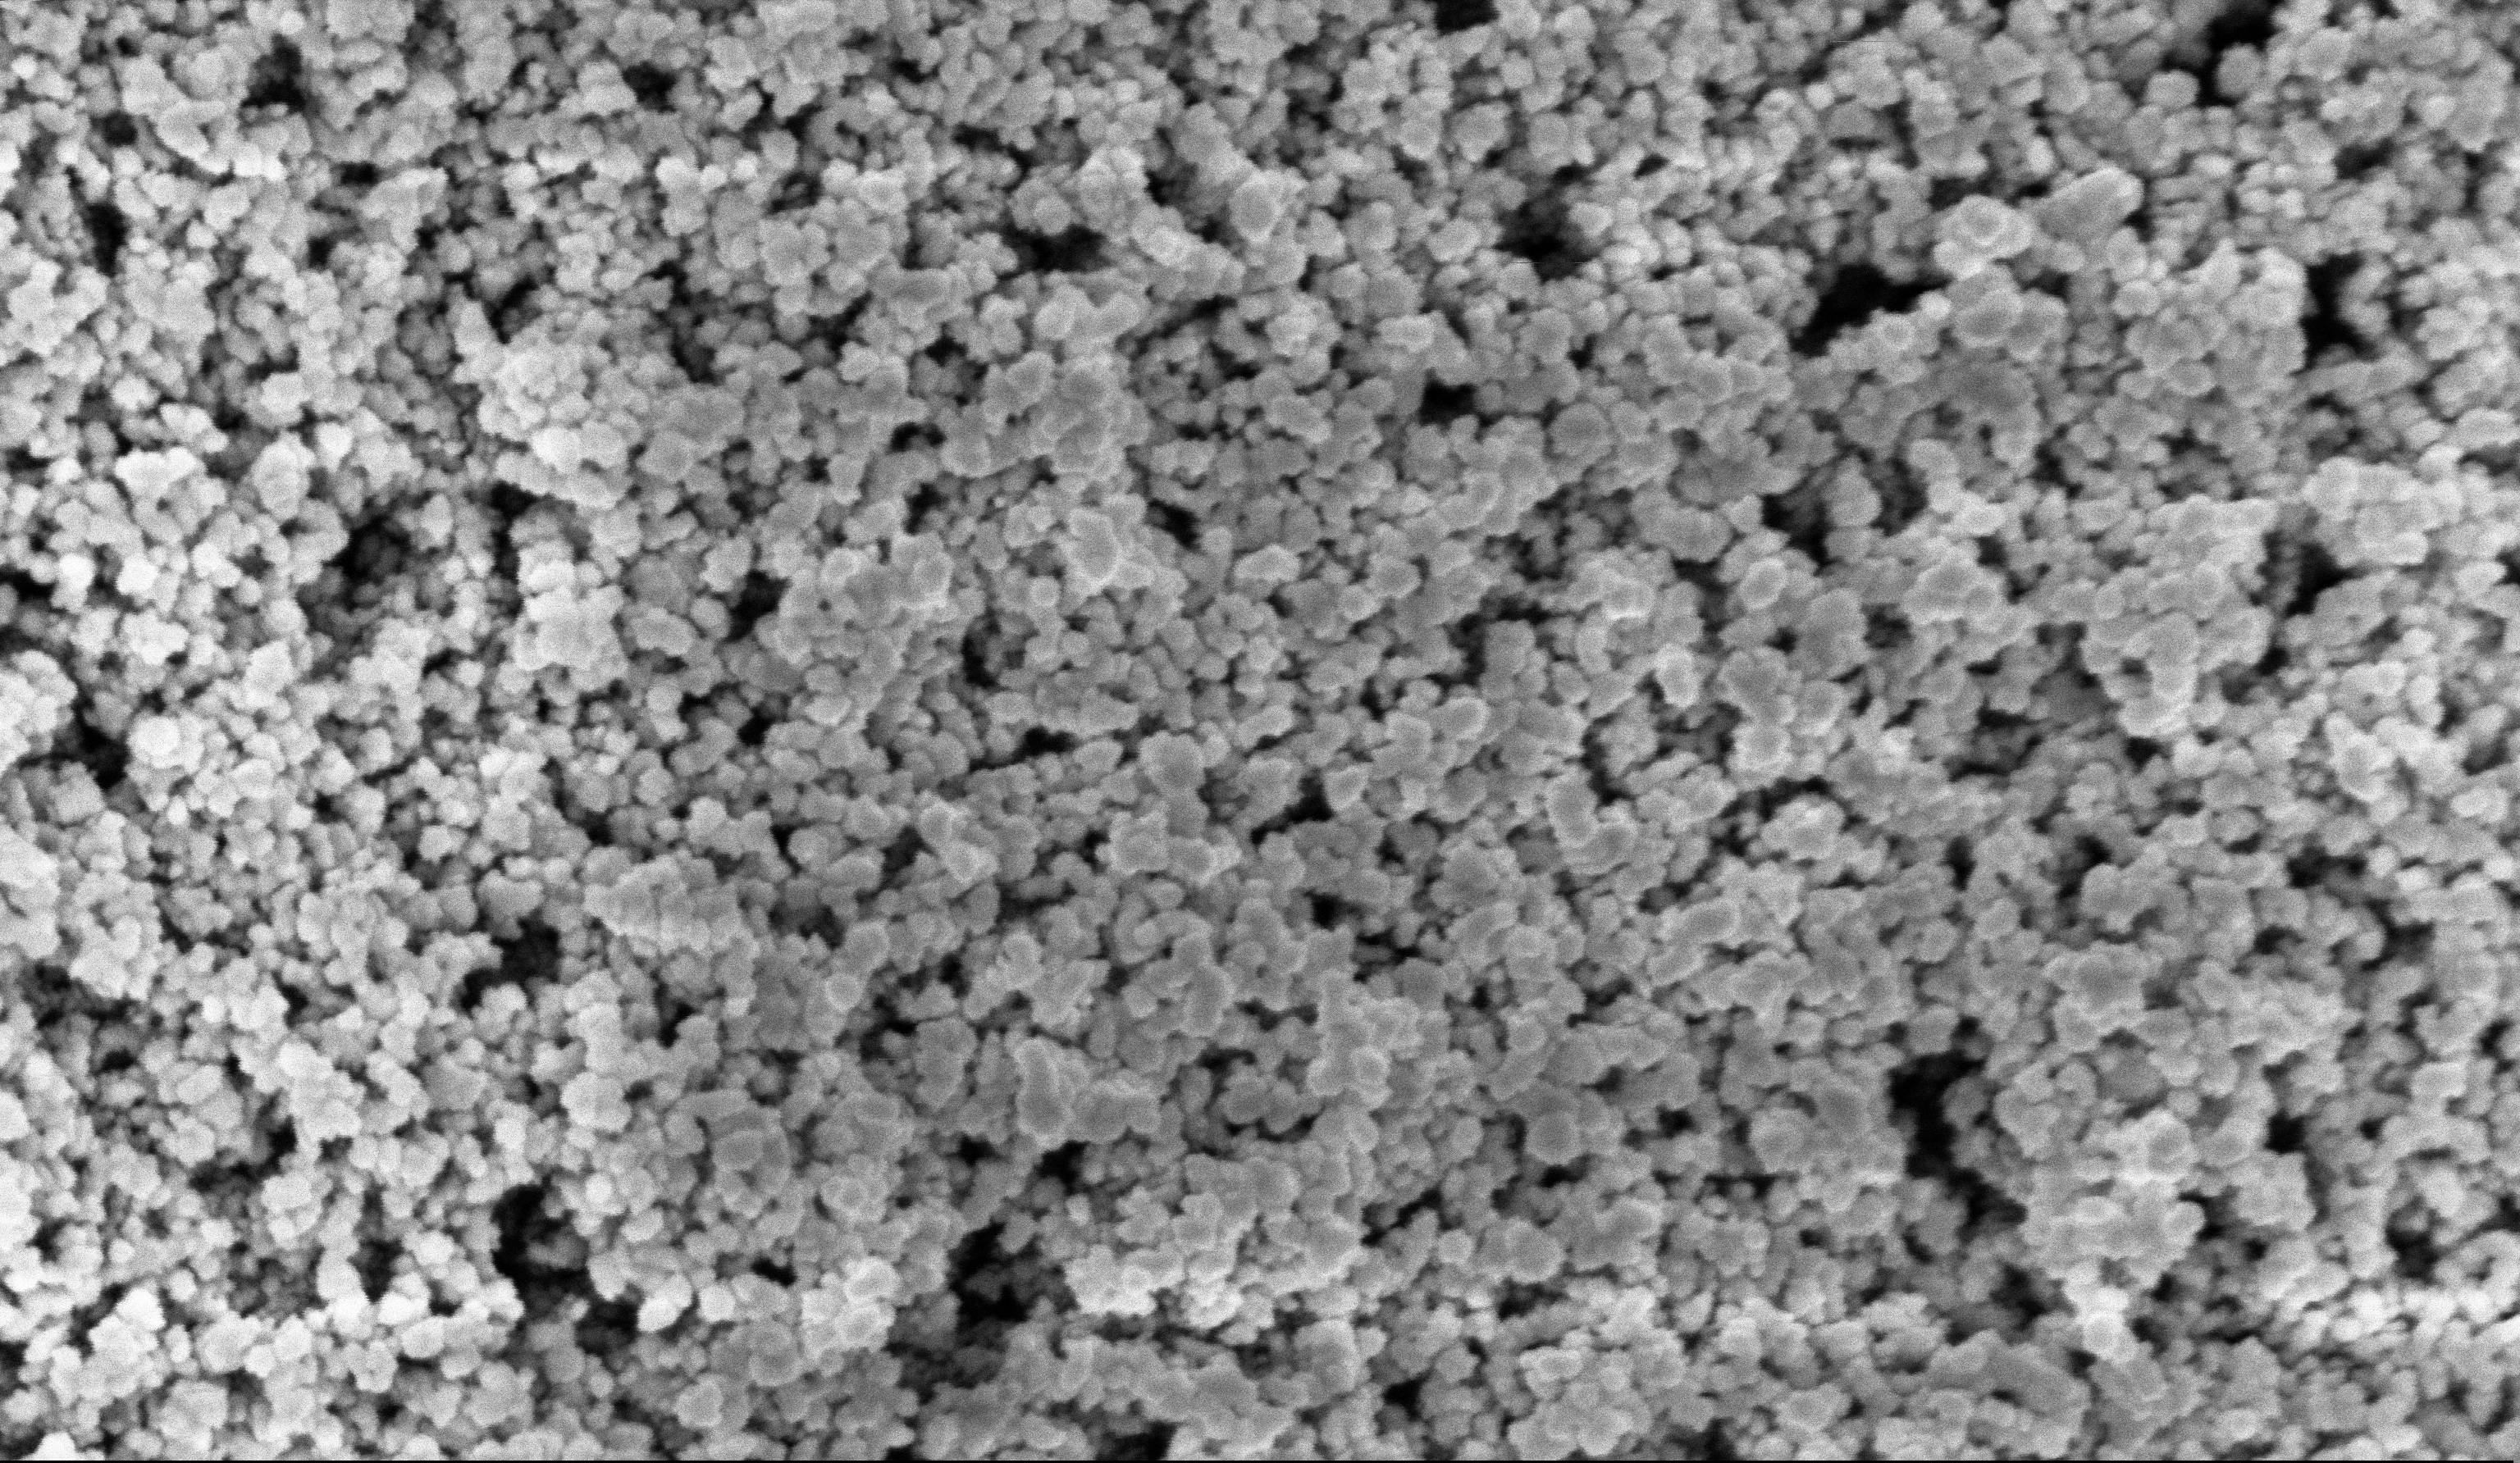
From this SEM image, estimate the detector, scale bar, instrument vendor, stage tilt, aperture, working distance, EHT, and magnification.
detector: InLens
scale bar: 100 nm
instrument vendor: Zeiss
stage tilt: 0°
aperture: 30 µm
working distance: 6 mm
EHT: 3 kV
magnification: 135 K X